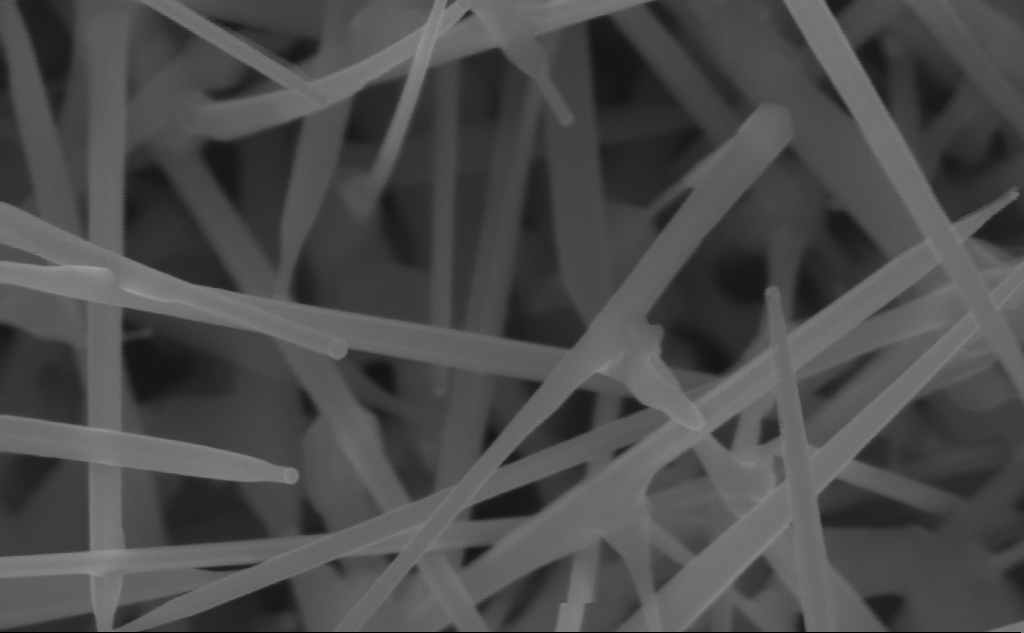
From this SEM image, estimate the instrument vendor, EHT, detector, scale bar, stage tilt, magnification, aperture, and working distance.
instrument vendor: Zeiss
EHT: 10 kV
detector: InLens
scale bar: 200 nm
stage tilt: -0°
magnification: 183.68 K X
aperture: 30 µm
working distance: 7 mm